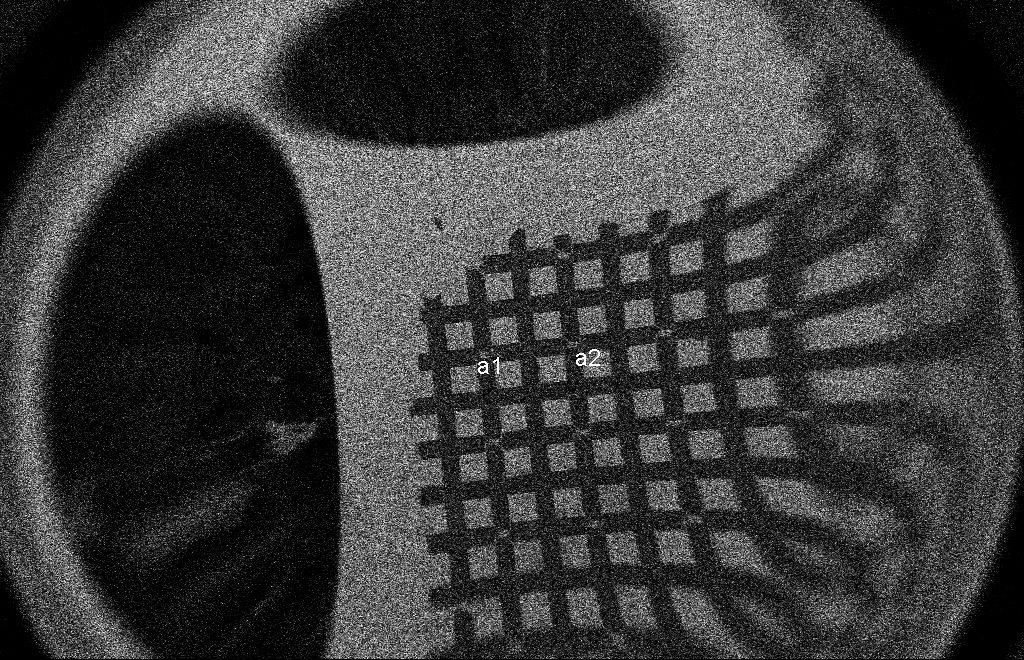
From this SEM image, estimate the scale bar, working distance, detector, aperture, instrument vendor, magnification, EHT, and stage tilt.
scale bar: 200000 nm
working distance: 9 mm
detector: SE2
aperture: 20 µm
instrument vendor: Zeiss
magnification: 0.067 K X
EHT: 2 kV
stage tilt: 0°